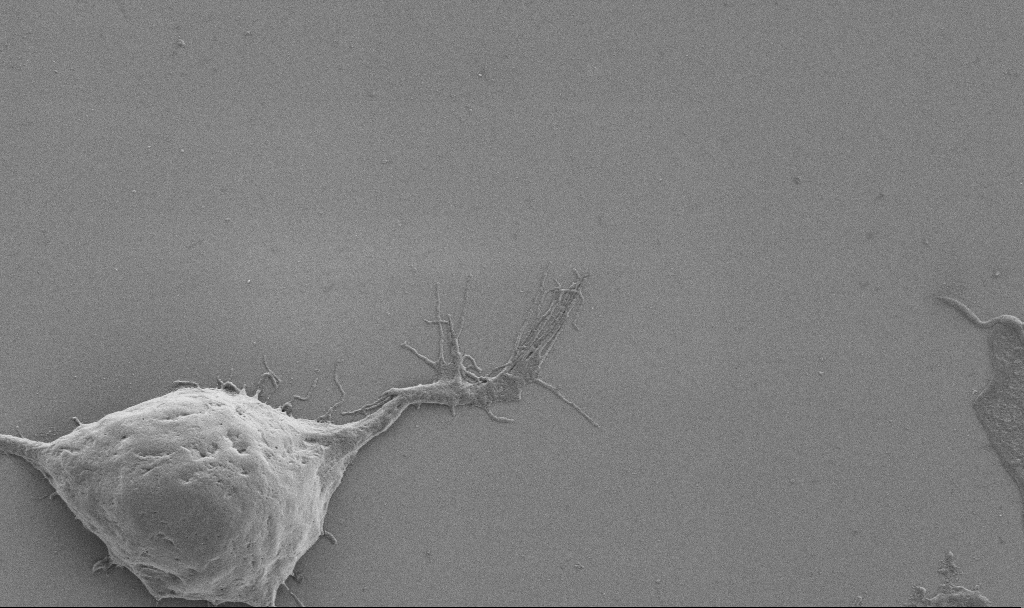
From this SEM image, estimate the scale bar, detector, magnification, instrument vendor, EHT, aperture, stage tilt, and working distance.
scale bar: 2000 nm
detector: SE2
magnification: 10 K X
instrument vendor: Zeiss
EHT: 0.9 kV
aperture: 30 µm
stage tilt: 0°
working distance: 6.9 mm